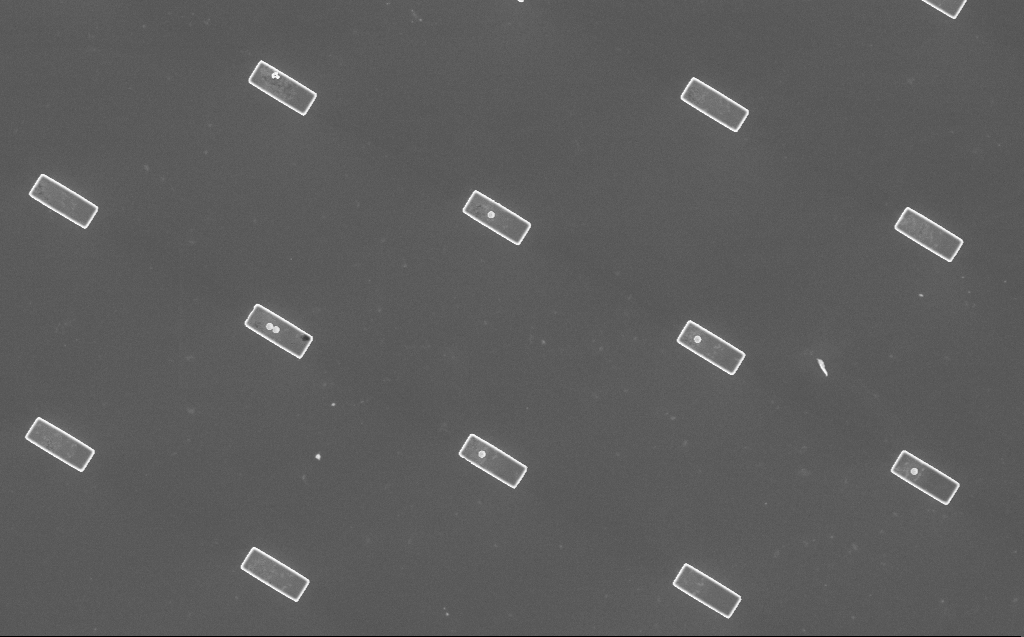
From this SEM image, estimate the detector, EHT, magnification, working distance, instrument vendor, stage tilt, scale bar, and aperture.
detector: InLens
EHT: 5 kV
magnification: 3.24 K X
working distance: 8 mm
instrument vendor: Zeiss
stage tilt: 0°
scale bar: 10000 nm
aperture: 30 µm